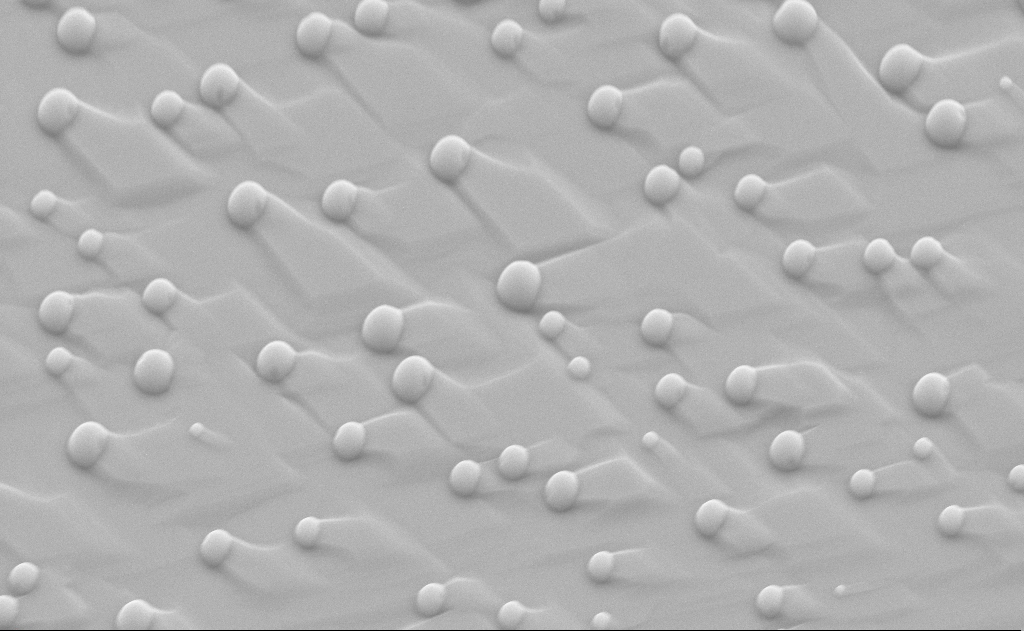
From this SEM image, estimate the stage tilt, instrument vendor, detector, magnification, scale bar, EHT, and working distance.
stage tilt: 48.9°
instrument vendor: Zeiss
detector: SE2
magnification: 13.93 K X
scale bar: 2000 nm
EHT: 10 kV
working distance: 7 mm